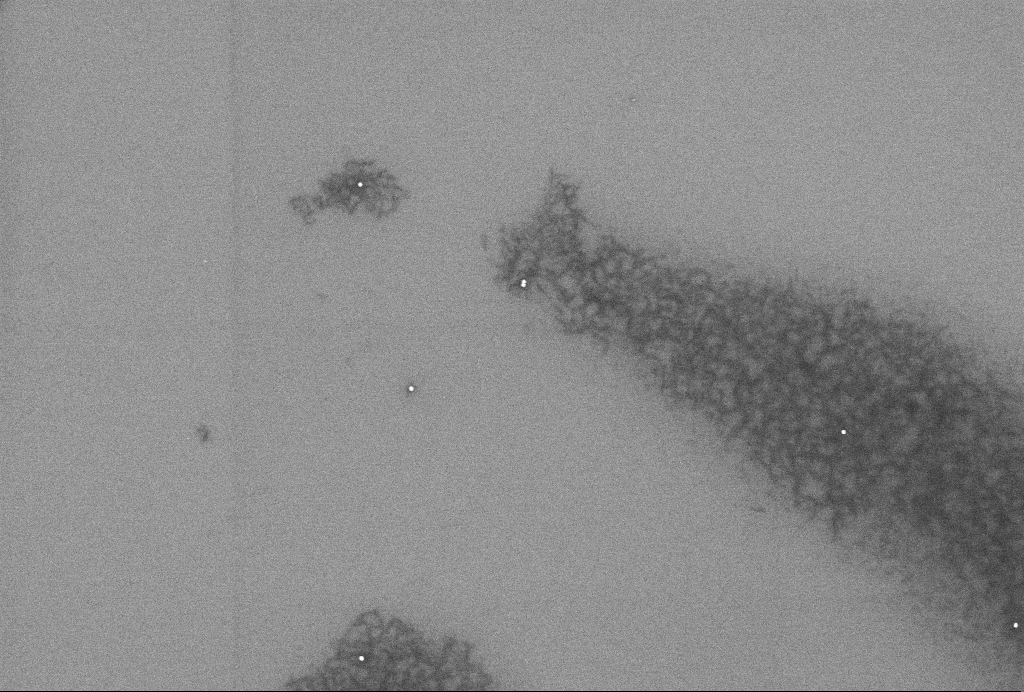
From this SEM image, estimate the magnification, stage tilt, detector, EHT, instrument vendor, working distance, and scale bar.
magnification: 55.18 K X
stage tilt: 0°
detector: InLens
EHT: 2 kV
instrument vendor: Zeiss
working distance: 3.3 mm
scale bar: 1000 nm